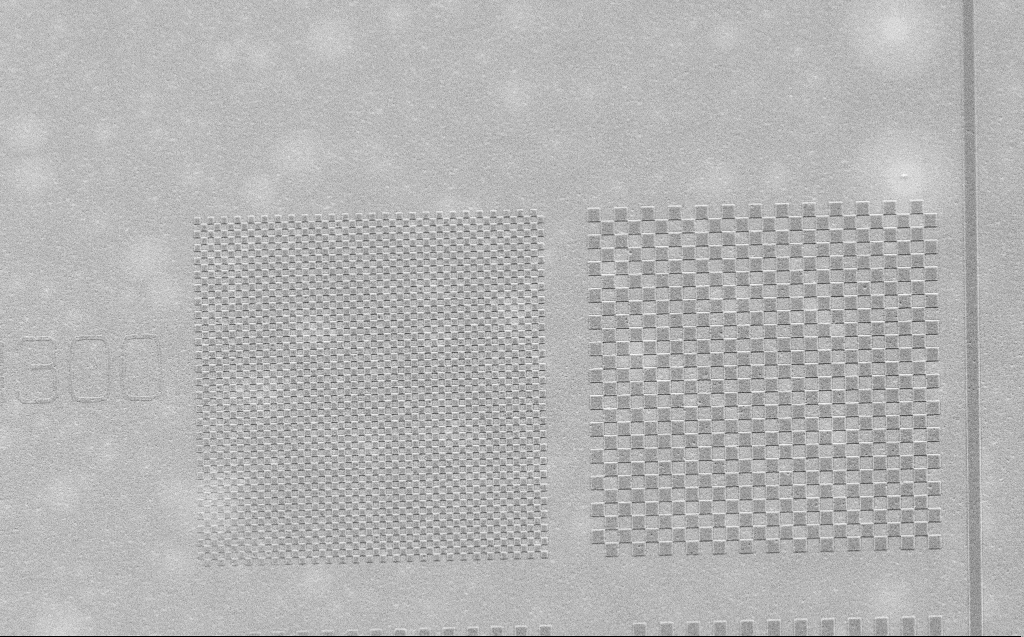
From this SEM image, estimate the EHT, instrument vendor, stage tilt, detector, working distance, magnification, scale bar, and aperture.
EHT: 2.5 kV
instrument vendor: Zeiss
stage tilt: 30°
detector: SE2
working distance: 4 mm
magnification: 5 K X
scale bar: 10000 nm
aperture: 30 µm